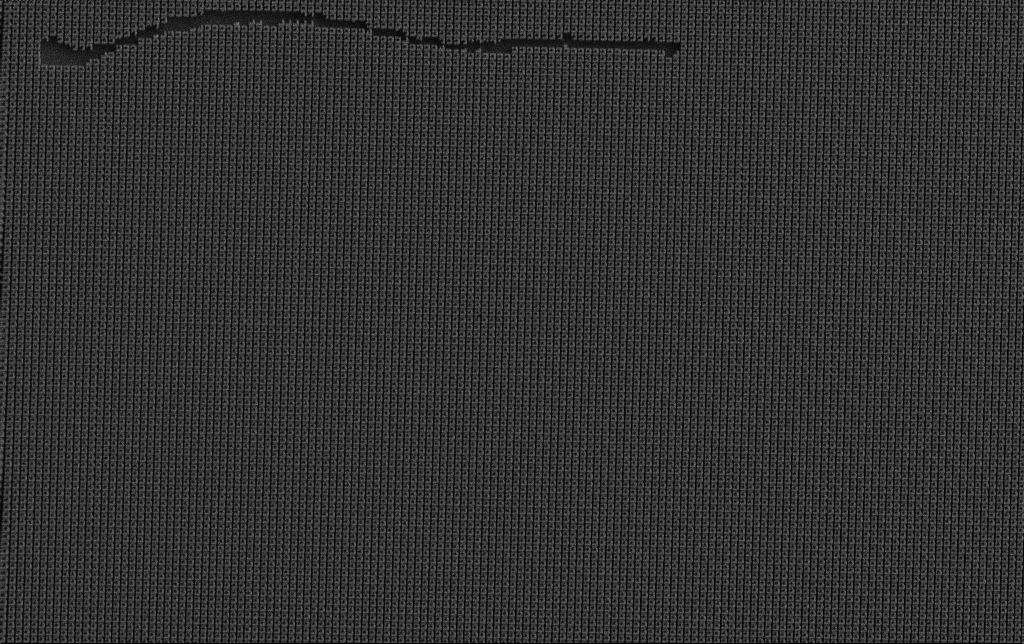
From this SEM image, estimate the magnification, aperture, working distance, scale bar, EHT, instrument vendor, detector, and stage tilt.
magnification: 5.89 K X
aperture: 30 µm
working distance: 3.3 mm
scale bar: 10000 nm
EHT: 5 kV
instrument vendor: Zeiss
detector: SE2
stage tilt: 0°